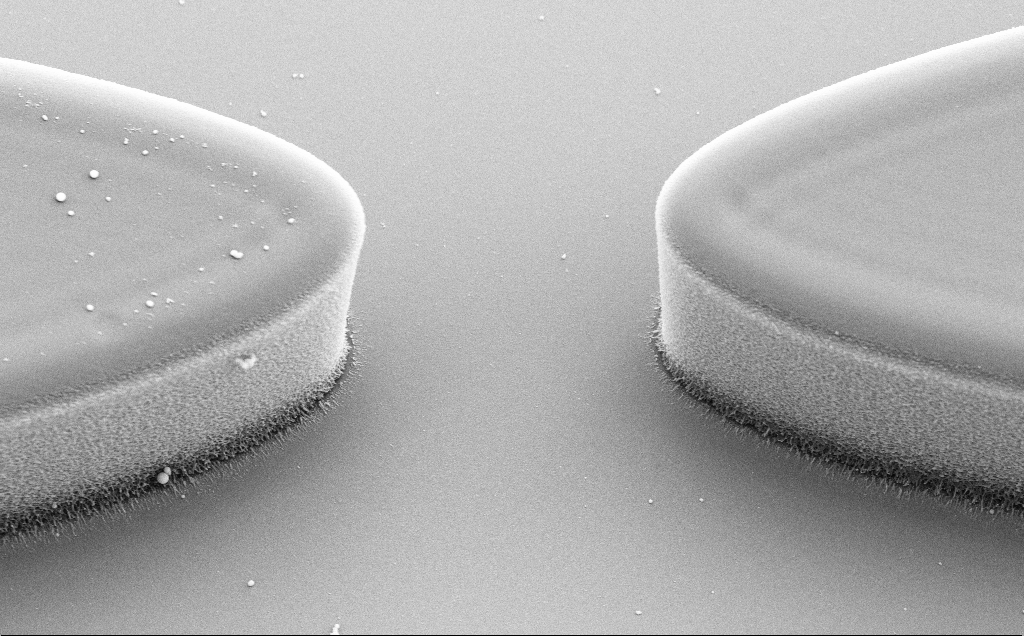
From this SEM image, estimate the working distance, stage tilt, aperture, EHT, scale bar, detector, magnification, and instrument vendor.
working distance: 8 mm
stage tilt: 30°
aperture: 30 µm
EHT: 5 kV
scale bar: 2000 nm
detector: SE2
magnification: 10.22 K X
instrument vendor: Zeiss